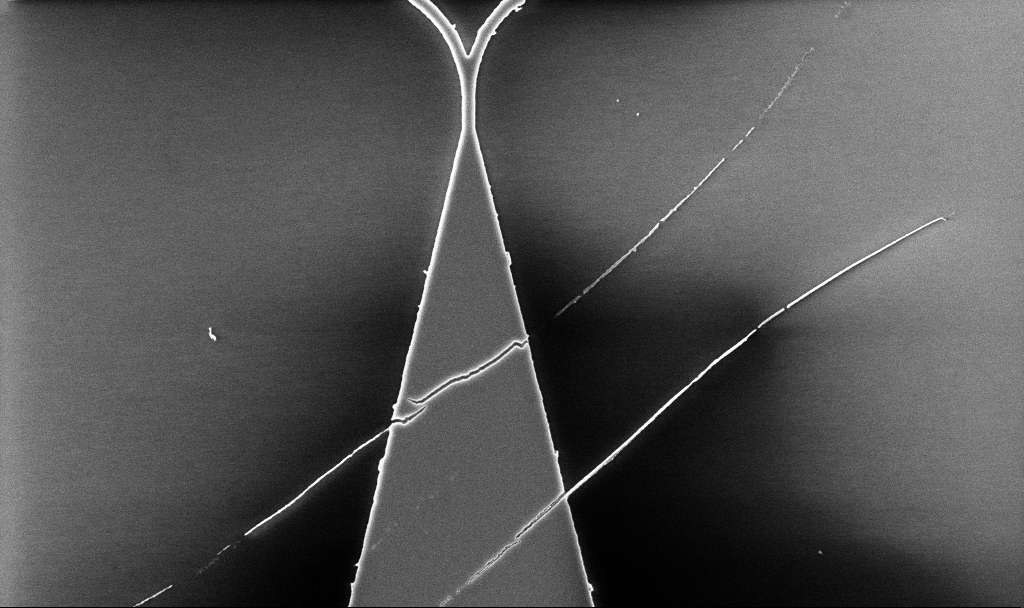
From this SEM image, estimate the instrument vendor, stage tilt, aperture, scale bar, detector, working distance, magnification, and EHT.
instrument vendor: Zeiss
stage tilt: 0°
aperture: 30 µm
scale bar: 2000 nm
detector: InLens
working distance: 5.2 mm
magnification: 9.78 K X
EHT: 5 kV